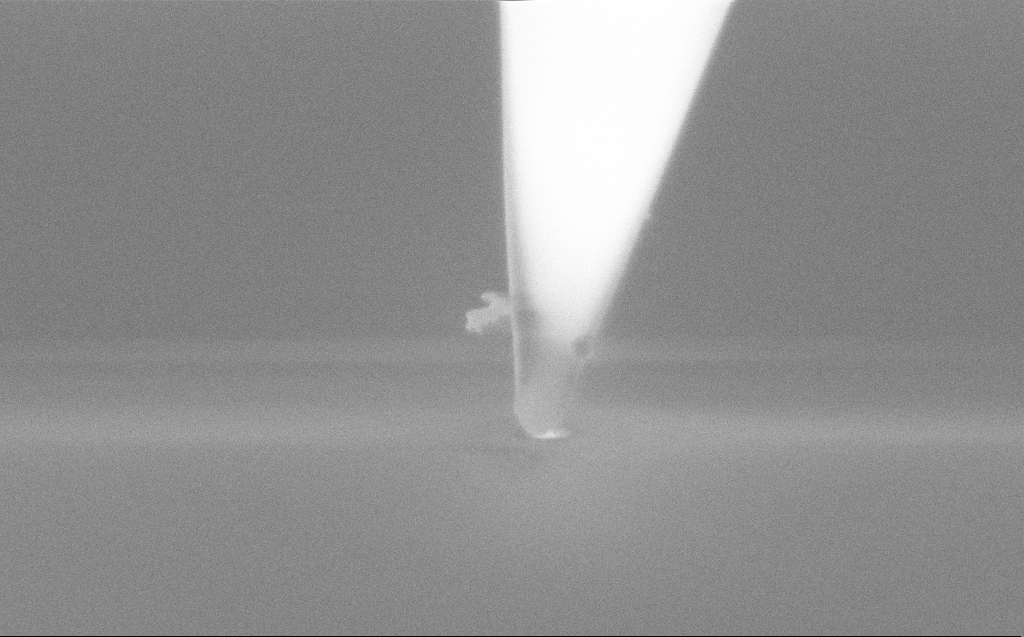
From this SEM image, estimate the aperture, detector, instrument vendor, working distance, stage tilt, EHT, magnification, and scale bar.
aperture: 30 µm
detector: InLens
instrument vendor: Zeiss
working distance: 5 mm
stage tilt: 45.1°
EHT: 3 kV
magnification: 146.02 K X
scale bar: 200 nm